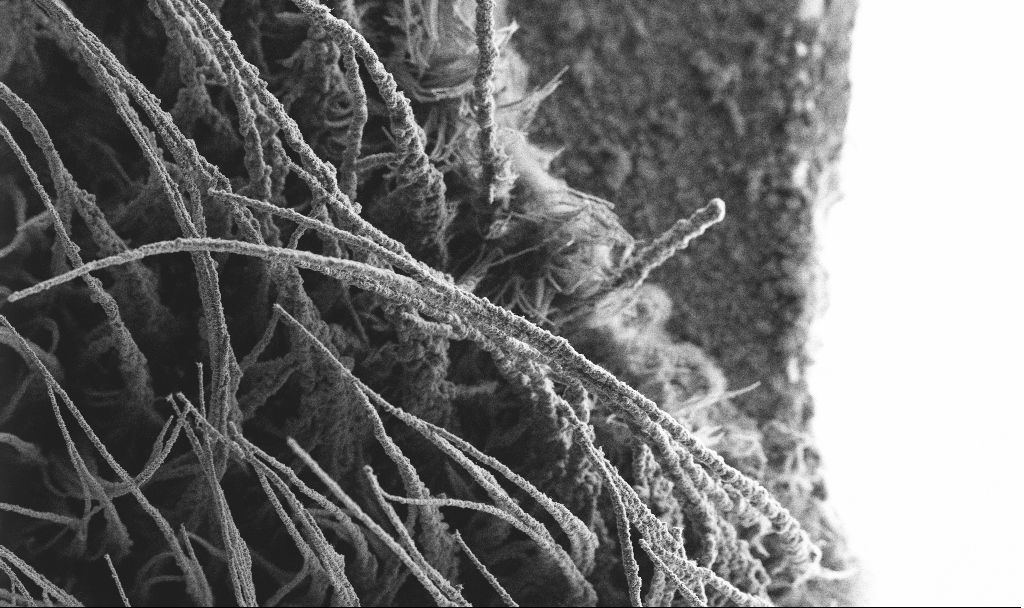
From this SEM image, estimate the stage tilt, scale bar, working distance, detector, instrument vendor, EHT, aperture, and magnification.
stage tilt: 0°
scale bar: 100000 nm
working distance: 2.9 mm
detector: SE2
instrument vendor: Zeiss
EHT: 3 kV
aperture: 30 µm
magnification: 0.15 K X